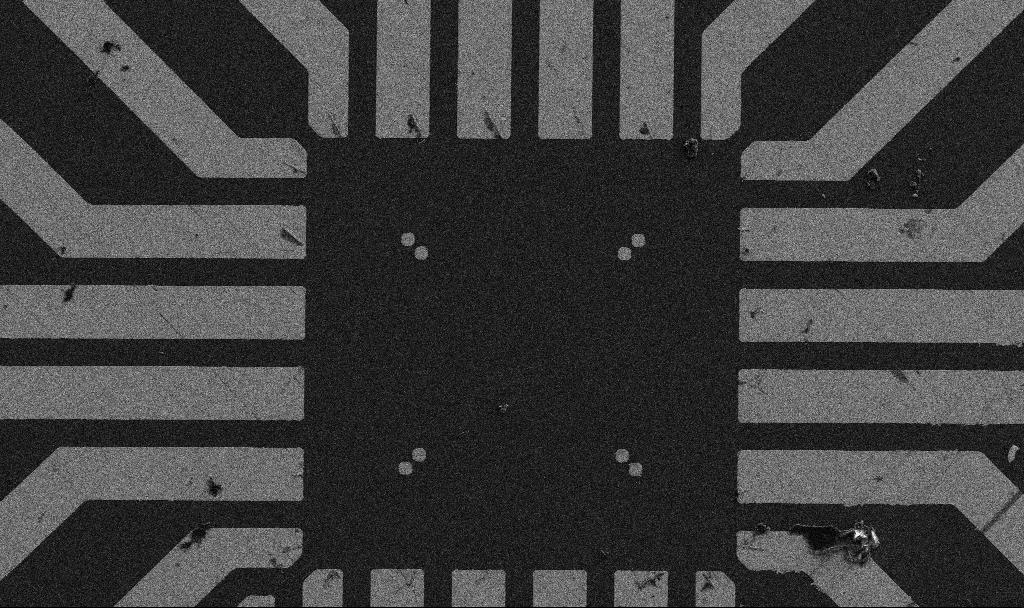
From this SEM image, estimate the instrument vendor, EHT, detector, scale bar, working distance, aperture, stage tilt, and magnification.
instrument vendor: Zeiss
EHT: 5 kV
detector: SE2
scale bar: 20000 nm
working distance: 9 mm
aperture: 30 µm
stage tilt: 0°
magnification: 1 K X